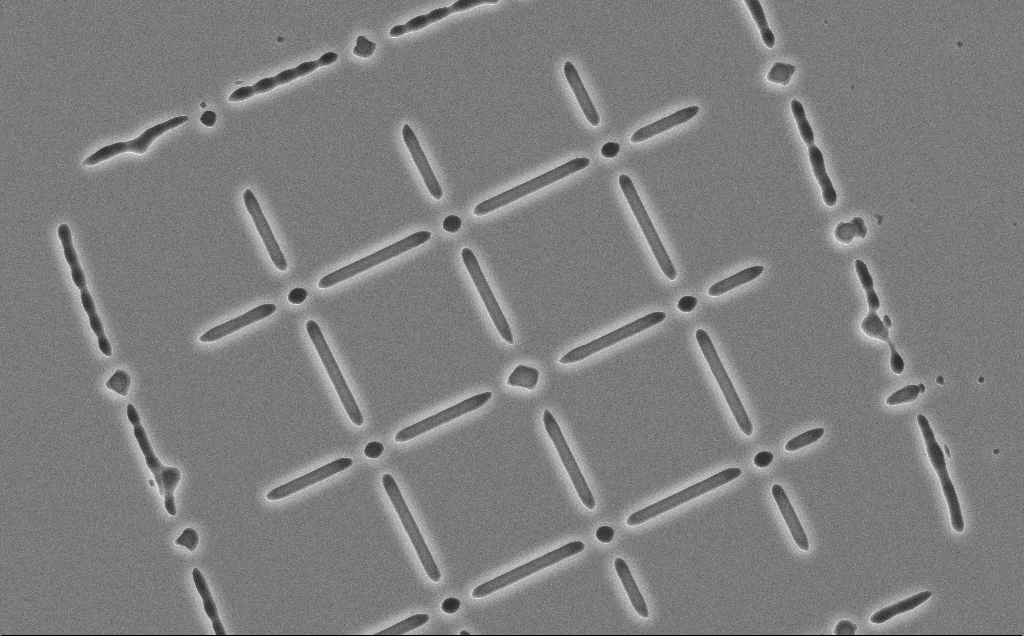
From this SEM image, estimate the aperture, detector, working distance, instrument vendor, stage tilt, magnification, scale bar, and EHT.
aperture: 30 µm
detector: SE2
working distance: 12 mm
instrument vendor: Zeiss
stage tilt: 0°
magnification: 3.14 K X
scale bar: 10000 nm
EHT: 10 kV